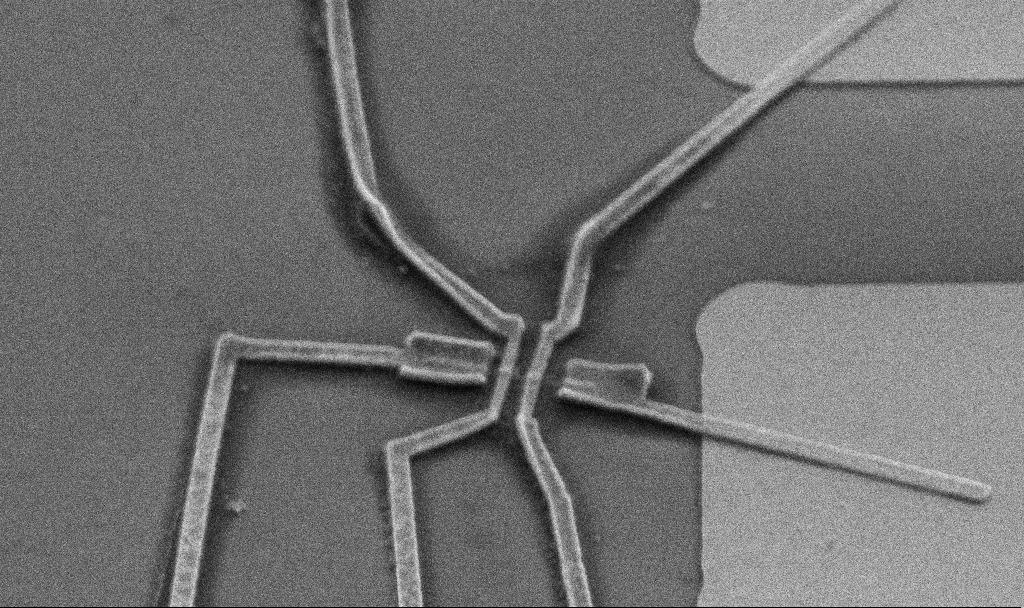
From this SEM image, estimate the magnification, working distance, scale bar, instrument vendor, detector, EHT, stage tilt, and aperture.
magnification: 10 K X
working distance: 12.4 mm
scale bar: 2000 nm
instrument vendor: Zeiss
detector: SE2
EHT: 5 kV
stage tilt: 45°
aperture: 30 µm